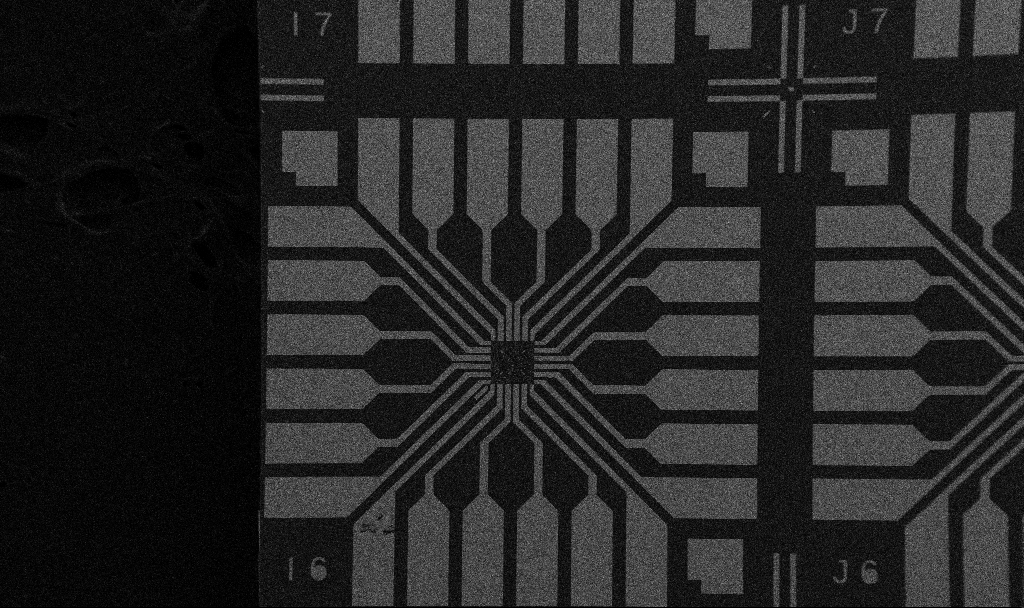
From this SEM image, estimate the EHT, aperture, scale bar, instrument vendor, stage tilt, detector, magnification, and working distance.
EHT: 5 kV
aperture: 30 µm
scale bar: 200000 nm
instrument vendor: Zeiss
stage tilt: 0°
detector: SE2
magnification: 0.1 K X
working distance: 10.7 mm